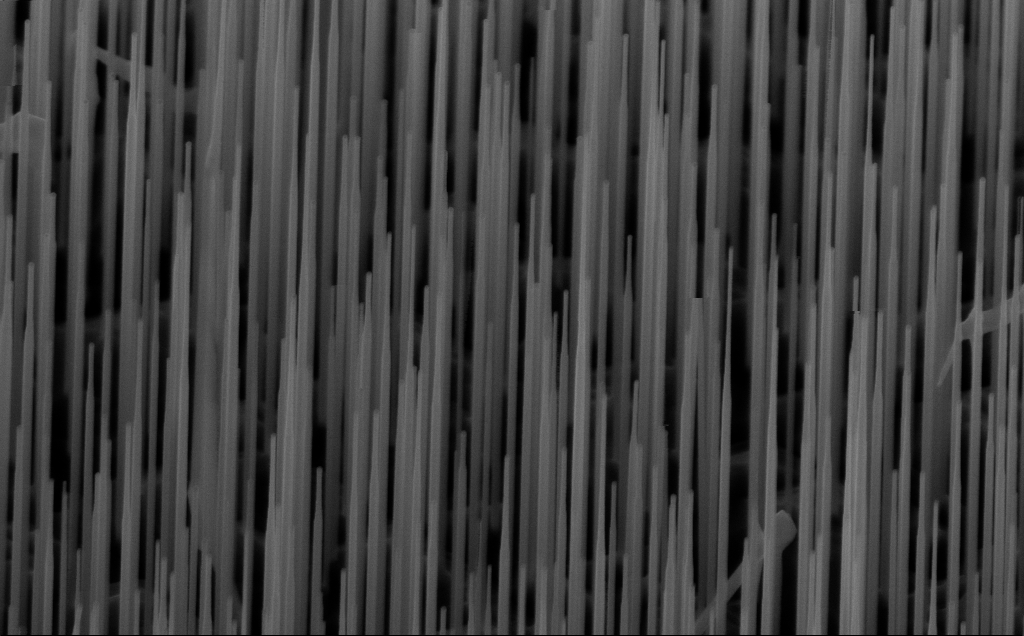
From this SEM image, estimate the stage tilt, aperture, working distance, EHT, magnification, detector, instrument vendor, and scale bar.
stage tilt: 45°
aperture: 30 µm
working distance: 6 mm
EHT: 10 kV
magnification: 40 K X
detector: InLens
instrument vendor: Zeiss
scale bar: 1000 nm